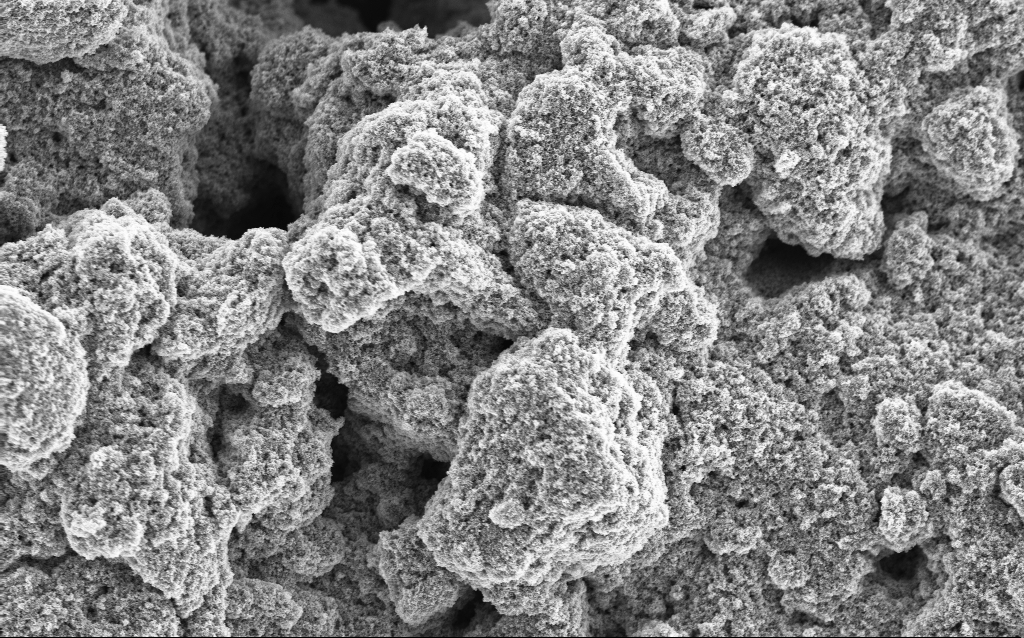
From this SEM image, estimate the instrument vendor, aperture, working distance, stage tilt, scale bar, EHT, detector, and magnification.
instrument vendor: Zeiss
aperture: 30 µm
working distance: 4.5 mm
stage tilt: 0°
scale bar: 10000 nm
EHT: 5 kV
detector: InLens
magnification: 6.4 K X